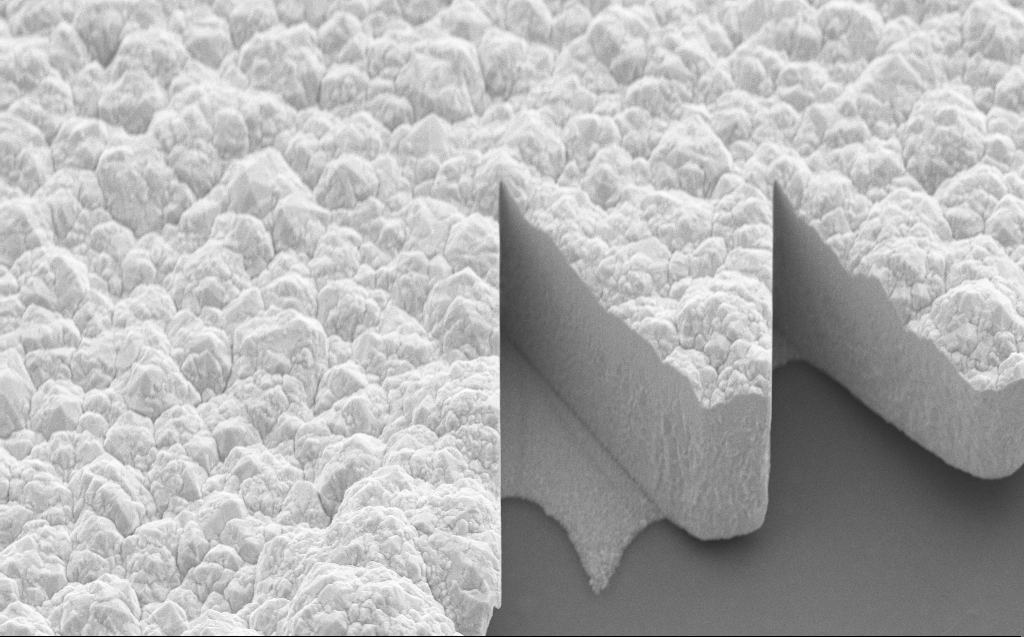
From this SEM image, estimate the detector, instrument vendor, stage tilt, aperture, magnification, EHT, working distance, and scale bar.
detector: SE2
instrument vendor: Zeiss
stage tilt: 45°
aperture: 30 µm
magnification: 8.73 K X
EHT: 10 kV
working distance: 9 mm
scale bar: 2000 nm